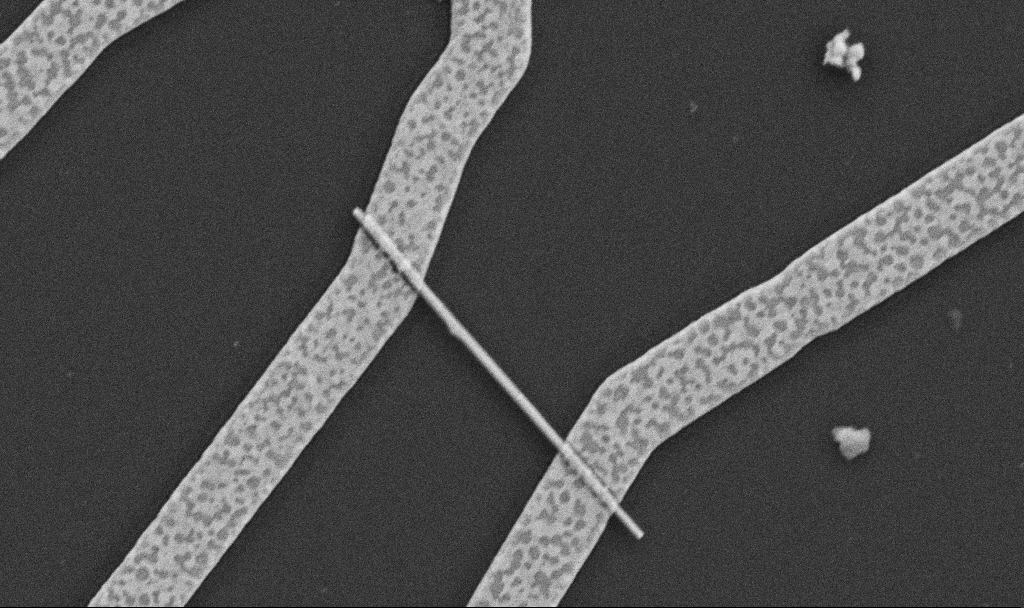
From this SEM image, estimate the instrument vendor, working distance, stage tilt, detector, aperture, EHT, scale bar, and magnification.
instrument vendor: Zeiss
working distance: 8.7 mm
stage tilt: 0°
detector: SE2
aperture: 30 µm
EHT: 5 kV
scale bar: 1000 nm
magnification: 30 K X